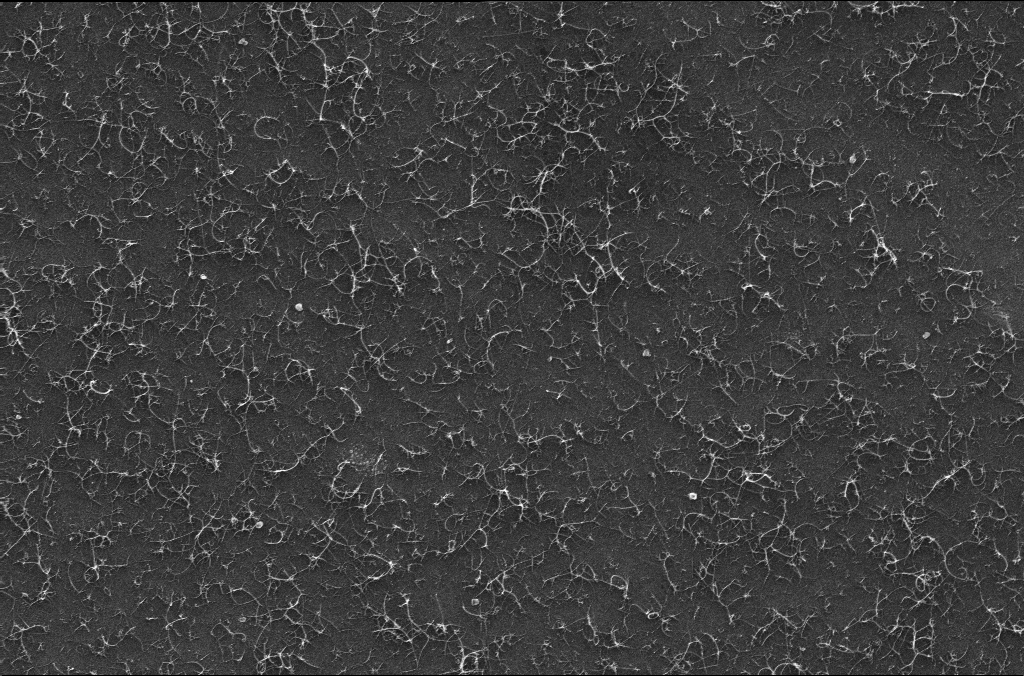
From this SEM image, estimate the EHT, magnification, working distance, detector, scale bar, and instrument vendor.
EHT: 10 kV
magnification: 34.87 K X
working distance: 3.2 mm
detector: InLens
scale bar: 1000 nm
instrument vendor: Zeiss